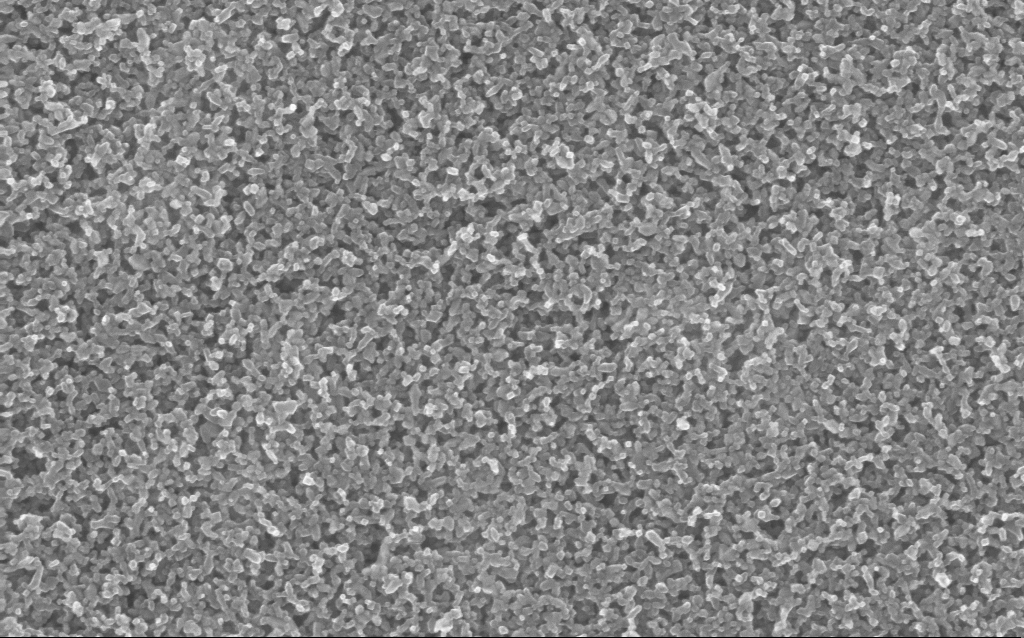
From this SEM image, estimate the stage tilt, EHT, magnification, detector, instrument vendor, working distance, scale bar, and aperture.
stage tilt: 0°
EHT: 10 kV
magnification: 130 K X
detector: InLens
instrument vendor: Zeiss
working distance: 5 mm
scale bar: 100 nm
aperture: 30 µm